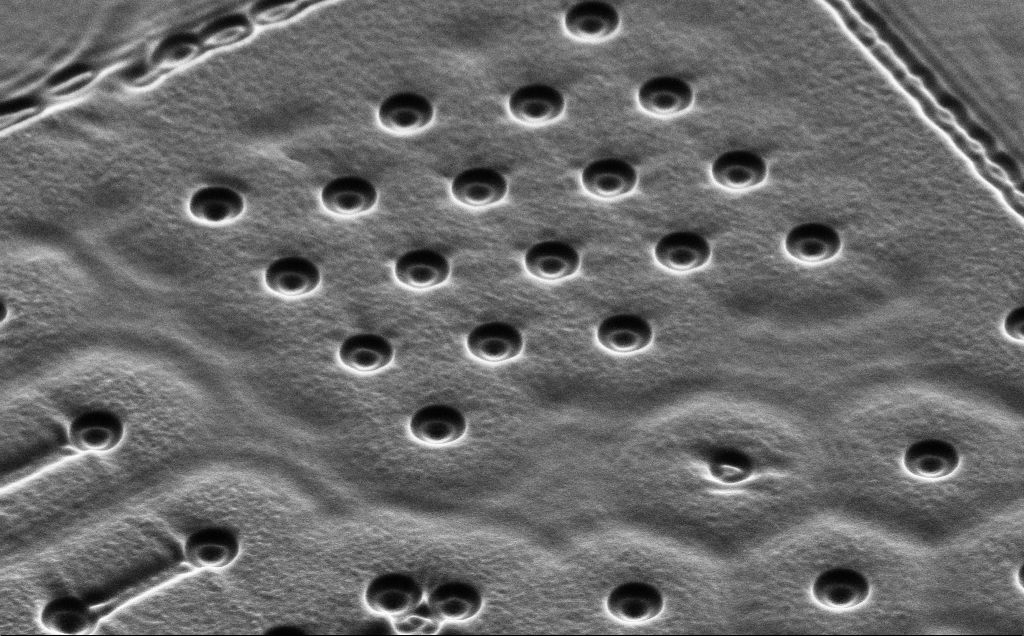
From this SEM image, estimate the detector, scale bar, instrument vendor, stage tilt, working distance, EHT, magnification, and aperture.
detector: SE2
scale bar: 2000 nm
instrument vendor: Zeiss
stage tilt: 45°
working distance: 10 mm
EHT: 5 kV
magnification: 7.53 K X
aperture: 30 µm